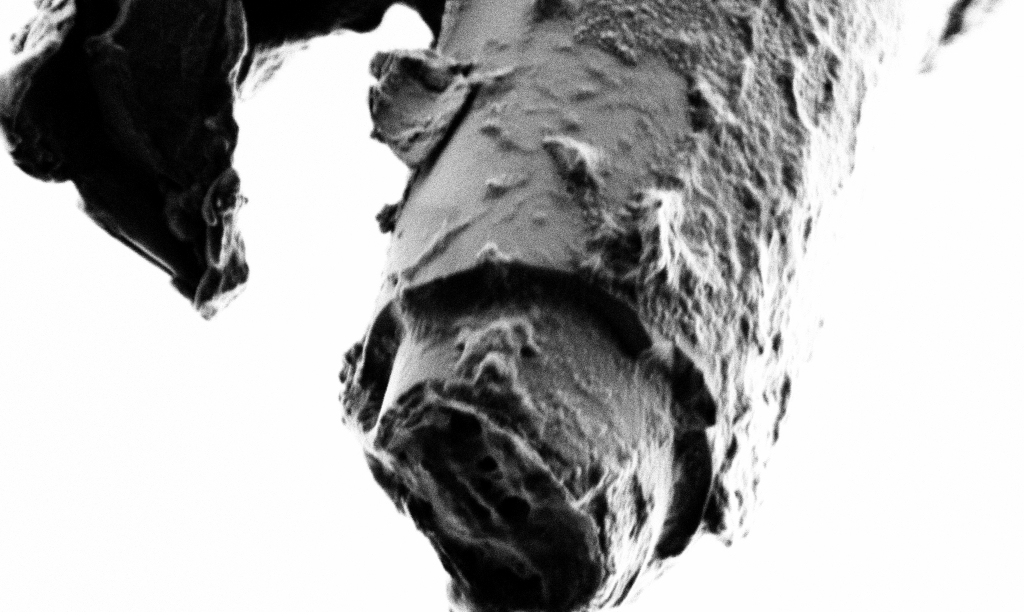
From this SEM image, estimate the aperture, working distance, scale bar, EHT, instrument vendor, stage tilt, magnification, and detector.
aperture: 30 µm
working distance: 4 mm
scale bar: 1000 nm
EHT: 1 kV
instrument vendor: Zeiss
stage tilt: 45°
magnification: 42.35 K X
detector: SE2